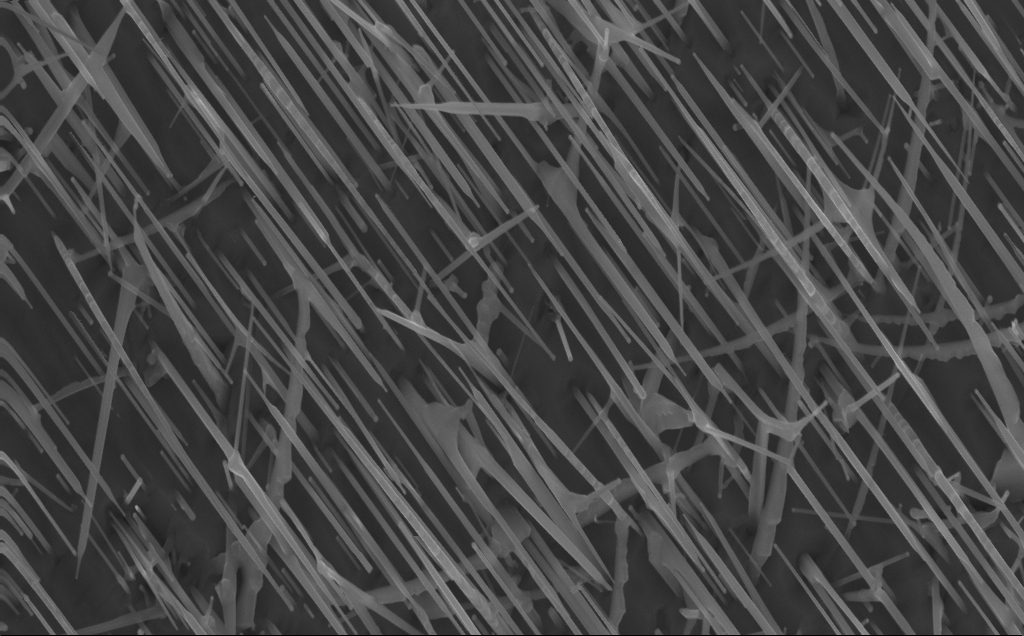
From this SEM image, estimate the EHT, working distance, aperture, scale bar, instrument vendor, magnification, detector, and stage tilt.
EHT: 10 kV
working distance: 4 mm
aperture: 30 µm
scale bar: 1000 nm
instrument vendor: Zeiss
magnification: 40 K X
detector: InLens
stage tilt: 0°